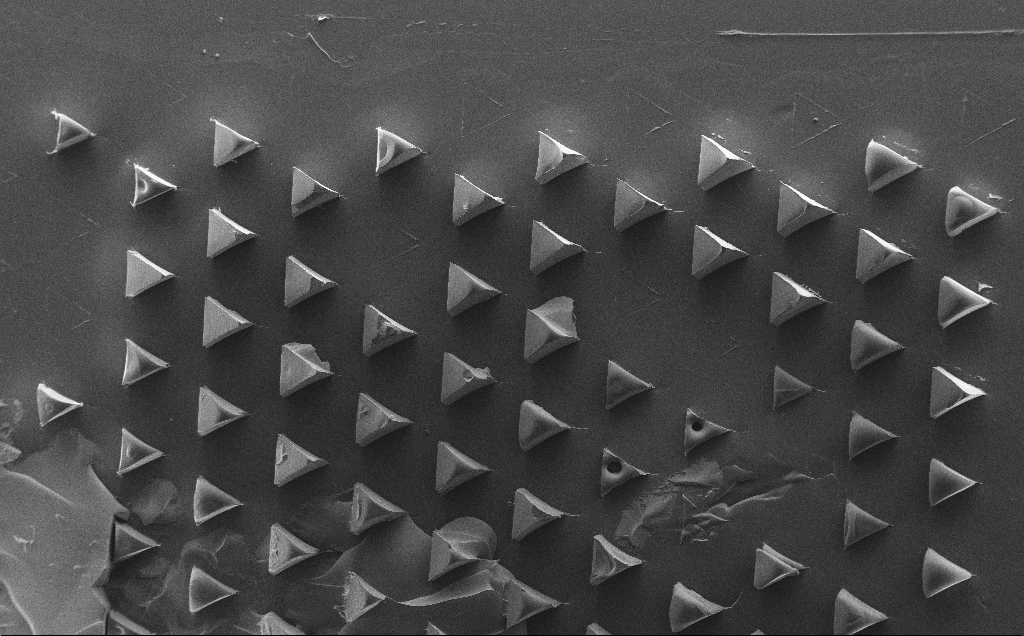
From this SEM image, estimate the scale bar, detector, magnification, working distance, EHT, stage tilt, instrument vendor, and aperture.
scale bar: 200000 nm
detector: SE2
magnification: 0.074 K X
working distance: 11 mm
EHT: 10 kV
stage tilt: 0°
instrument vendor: Zeiss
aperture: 30 µm